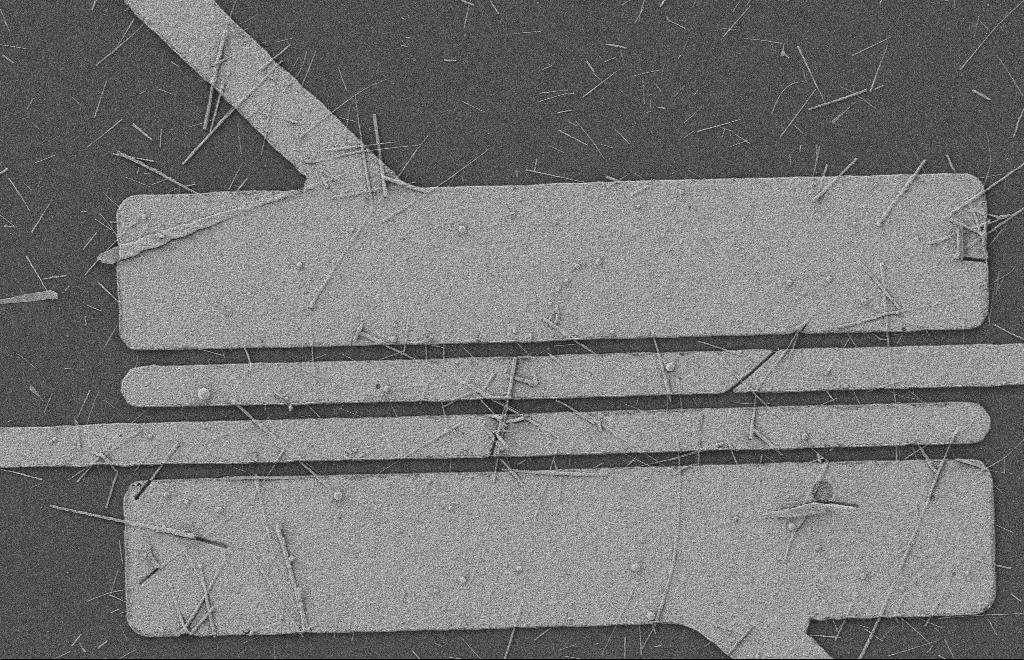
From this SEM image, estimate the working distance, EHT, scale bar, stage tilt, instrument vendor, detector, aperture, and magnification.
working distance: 8 mm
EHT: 2 kV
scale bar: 2000 nm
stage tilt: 0°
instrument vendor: Zeiss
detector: SE2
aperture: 20 µm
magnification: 5.22 K X